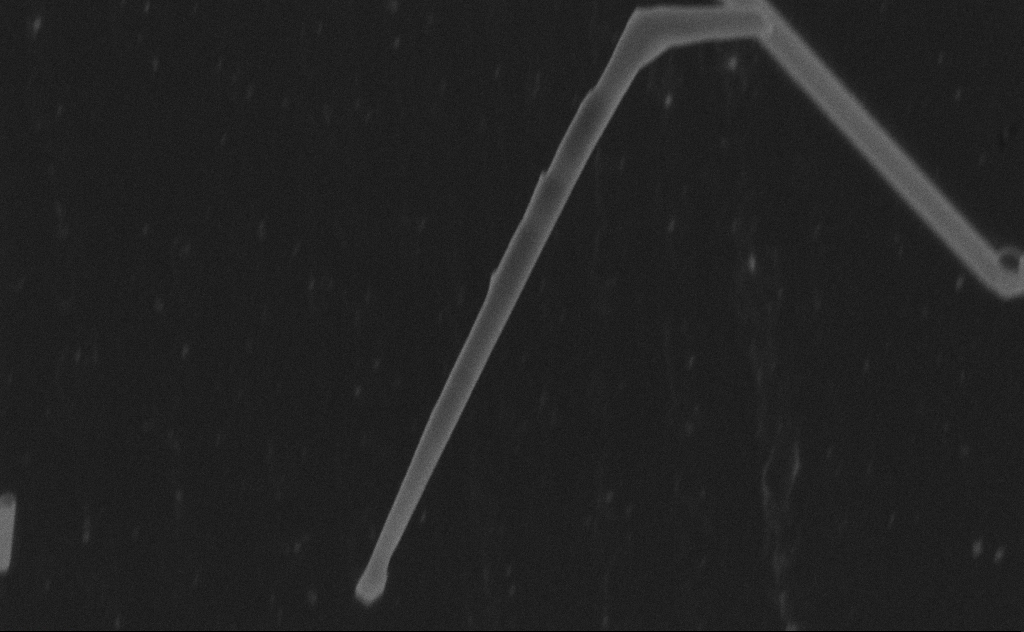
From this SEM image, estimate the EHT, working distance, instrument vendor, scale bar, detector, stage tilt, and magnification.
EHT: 20 kV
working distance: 9 mm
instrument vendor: Zeiss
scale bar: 200 nm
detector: SE2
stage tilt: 0°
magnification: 92.13 K X